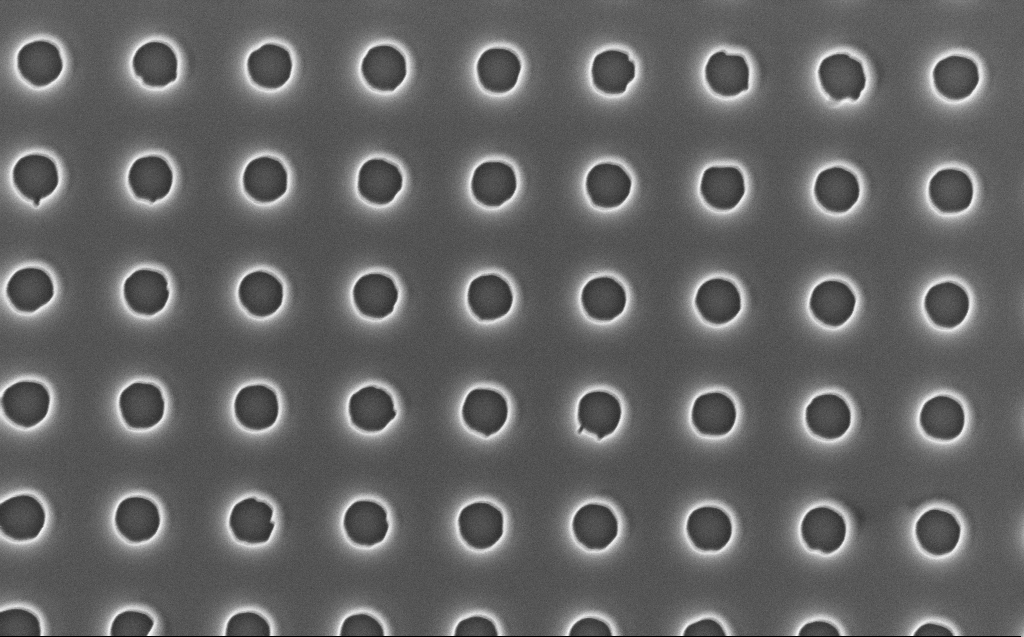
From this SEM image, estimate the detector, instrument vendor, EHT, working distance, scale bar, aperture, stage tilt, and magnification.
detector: InLens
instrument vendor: Zeiss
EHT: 5 kV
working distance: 7 mm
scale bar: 1000 nm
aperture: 30 µm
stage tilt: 0°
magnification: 40 K X